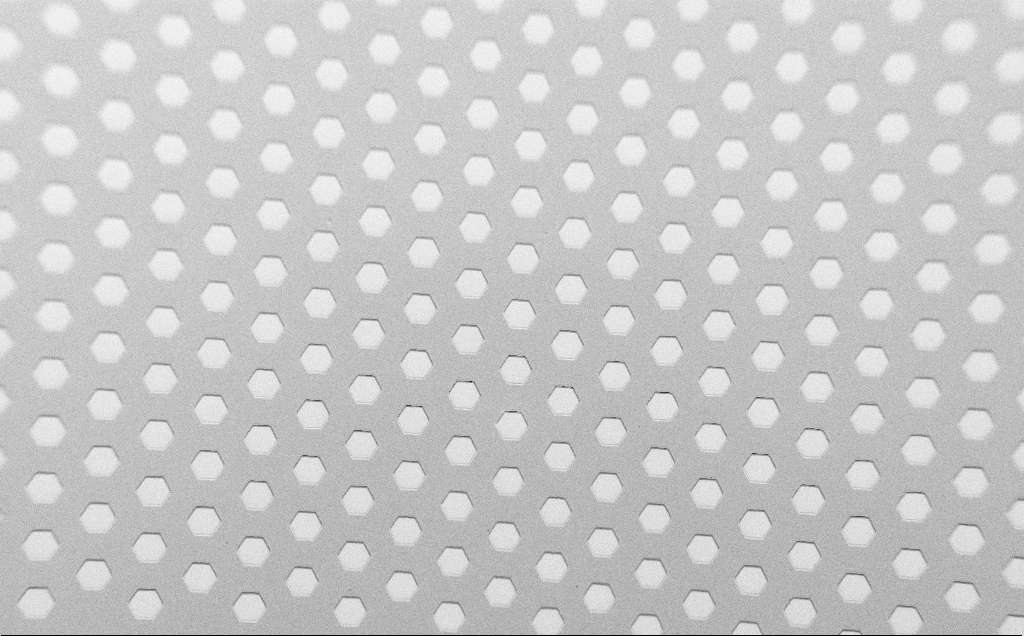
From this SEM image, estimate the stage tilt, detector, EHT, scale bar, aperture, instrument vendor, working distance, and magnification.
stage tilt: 45°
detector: SE2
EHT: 1.5 kV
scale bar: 100000 nm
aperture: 30 µm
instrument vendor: Zeiss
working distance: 6 mm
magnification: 0.214 K X